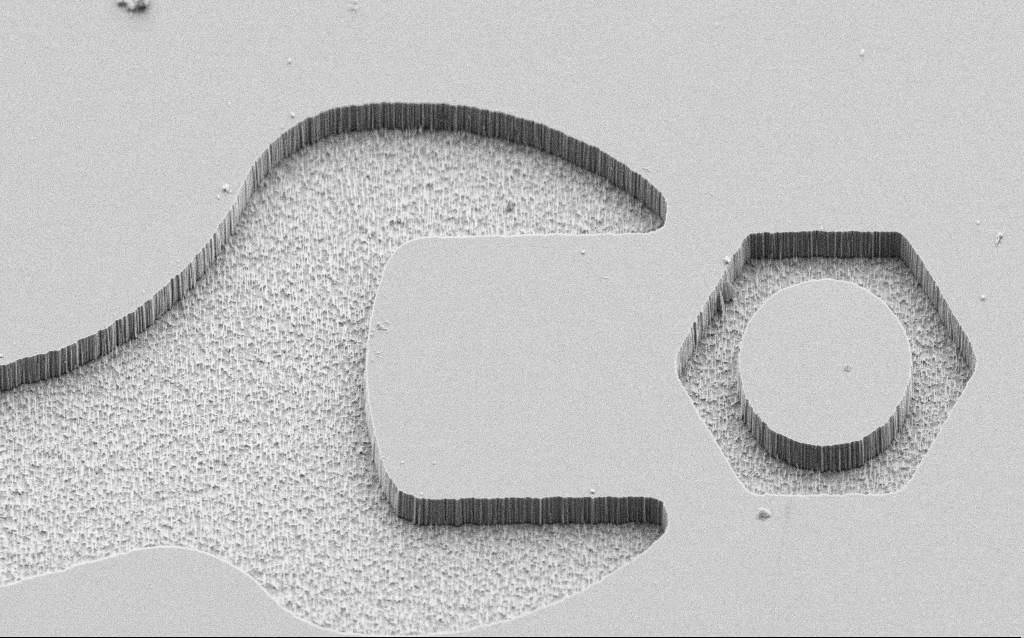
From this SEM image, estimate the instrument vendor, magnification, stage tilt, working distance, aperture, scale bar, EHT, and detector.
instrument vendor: Zeiss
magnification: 2 K X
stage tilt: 45°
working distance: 7 mm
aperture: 30 µm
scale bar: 20000 nm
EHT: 5 kV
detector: SE2